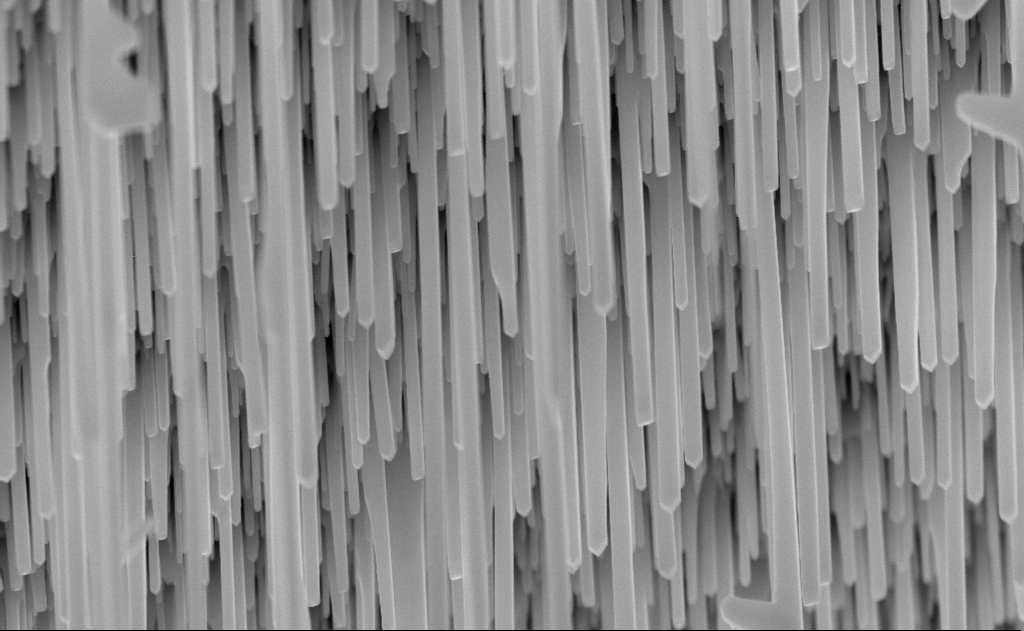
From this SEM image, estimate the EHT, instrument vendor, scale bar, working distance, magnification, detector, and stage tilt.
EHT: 10 kV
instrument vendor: Zeiss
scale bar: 1000 nm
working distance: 6 mm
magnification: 60 K X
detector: InLens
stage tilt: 0°